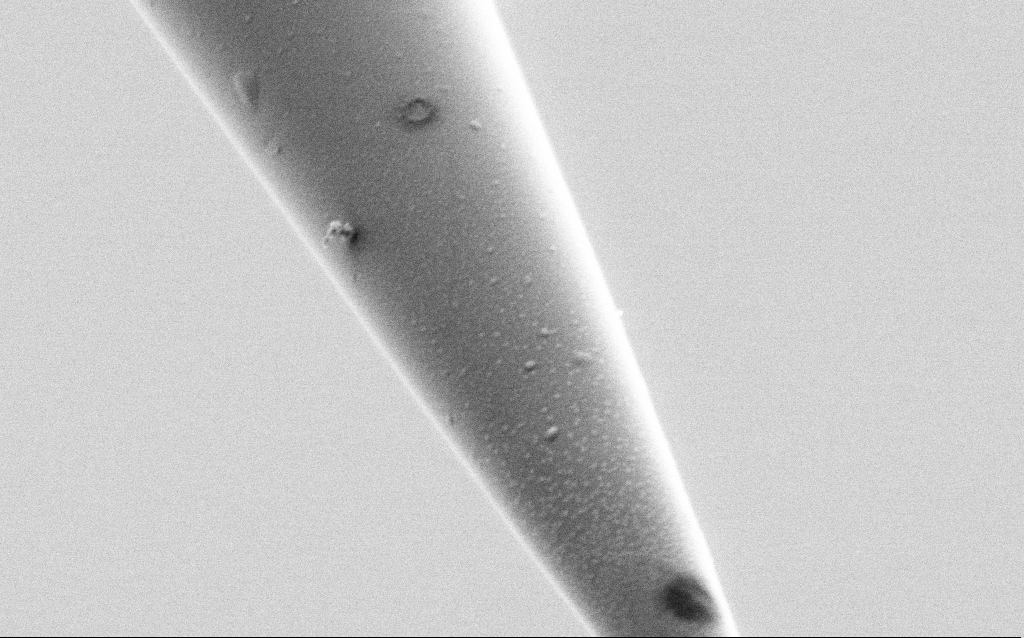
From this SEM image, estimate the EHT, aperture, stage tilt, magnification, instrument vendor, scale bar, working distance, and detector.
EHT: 1 kV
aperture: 30 µm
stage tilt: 45°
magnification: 50 K X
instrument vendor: Zeiss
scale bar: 1000 nm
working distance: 7.4 mm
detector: SE2